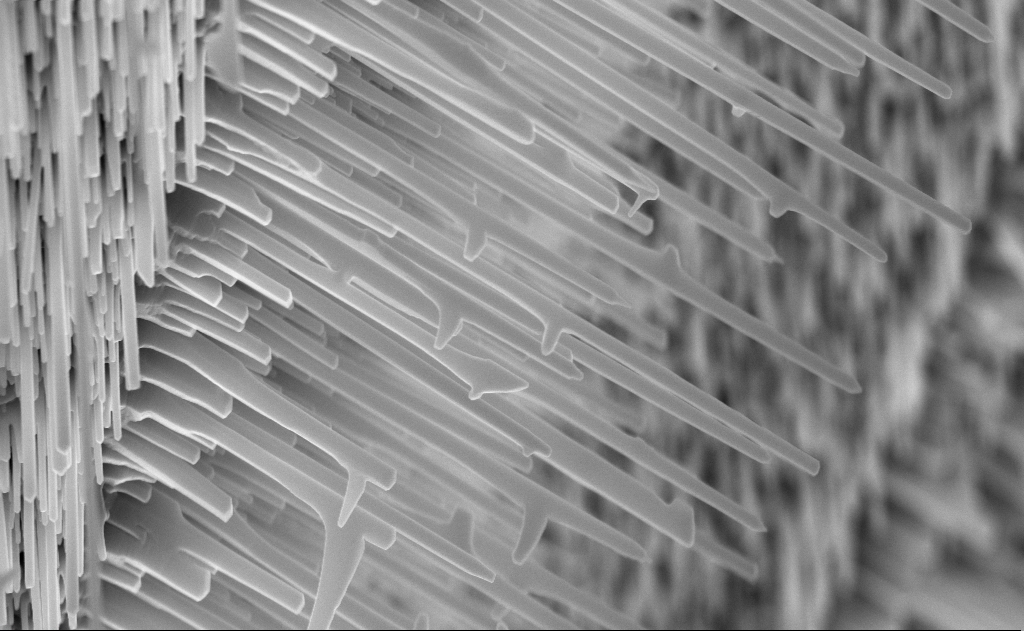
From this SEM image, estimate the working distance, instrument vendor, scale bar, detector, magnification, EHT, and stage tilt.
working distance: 6 mm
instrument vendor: Zeiss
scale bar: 1000 nm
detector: InLens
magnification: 40 K X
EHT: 10 kV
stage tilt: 0°